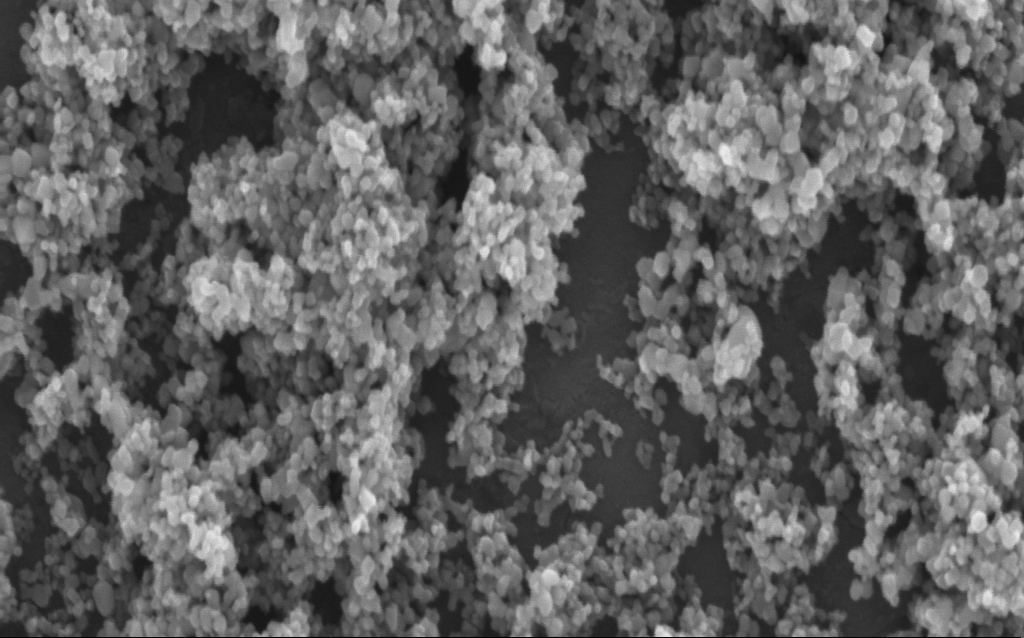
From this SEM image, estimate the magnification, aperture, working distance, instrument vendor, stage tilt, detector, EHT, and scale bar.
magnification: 138.82 K X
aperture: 30 µm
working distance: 4.7 mm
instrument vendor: Zeiss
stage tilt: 0°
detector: InLens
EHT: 5 kV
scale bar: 200 nm